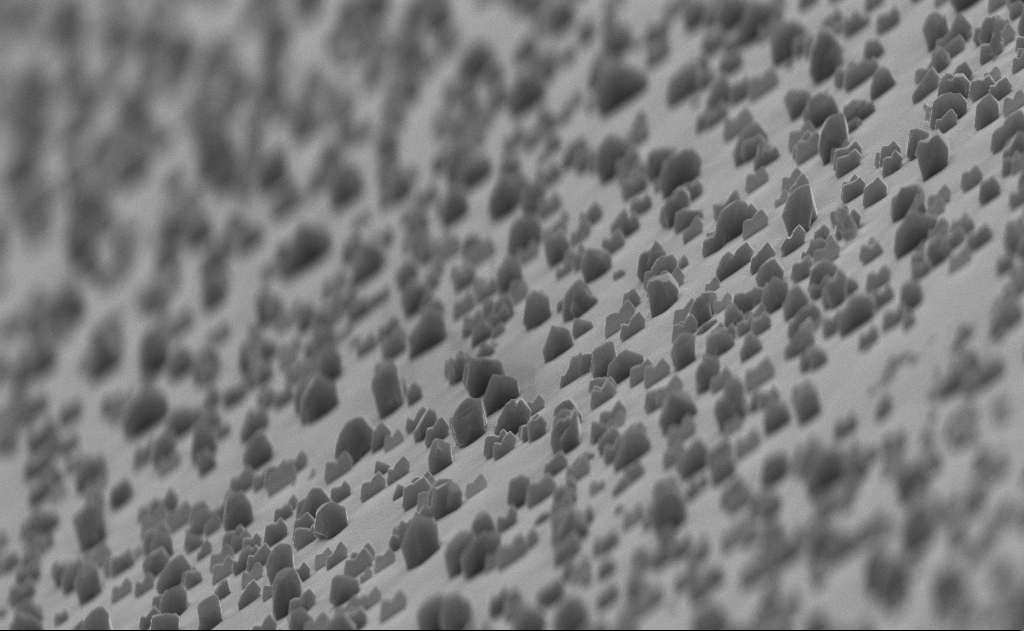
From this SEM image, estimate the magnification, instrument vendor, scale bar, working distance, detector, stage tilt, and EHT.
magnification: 20 K X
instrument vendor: Zeiss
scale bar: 2000 nm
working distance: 12 mm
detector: InLens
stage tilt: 0°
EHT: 10 kV